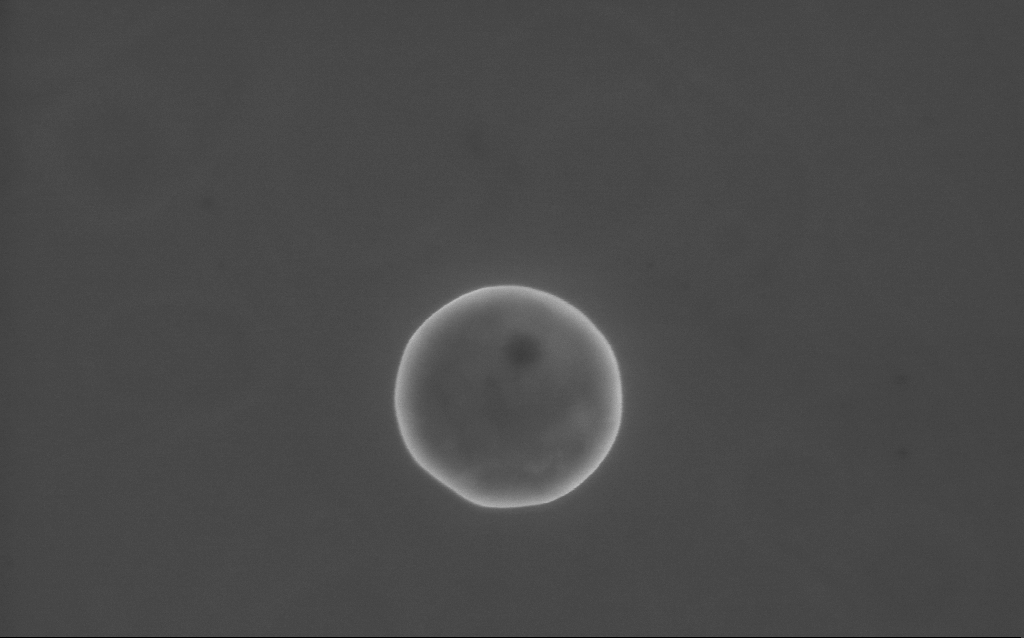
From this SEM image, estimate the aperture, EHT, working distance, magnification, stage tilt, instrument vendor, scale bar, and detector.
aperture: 30 µm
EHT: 10 kV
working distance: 4 mm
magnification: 65.43 K X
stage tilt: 0°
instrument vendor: Zeiss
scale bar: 1000 nm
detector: InLens